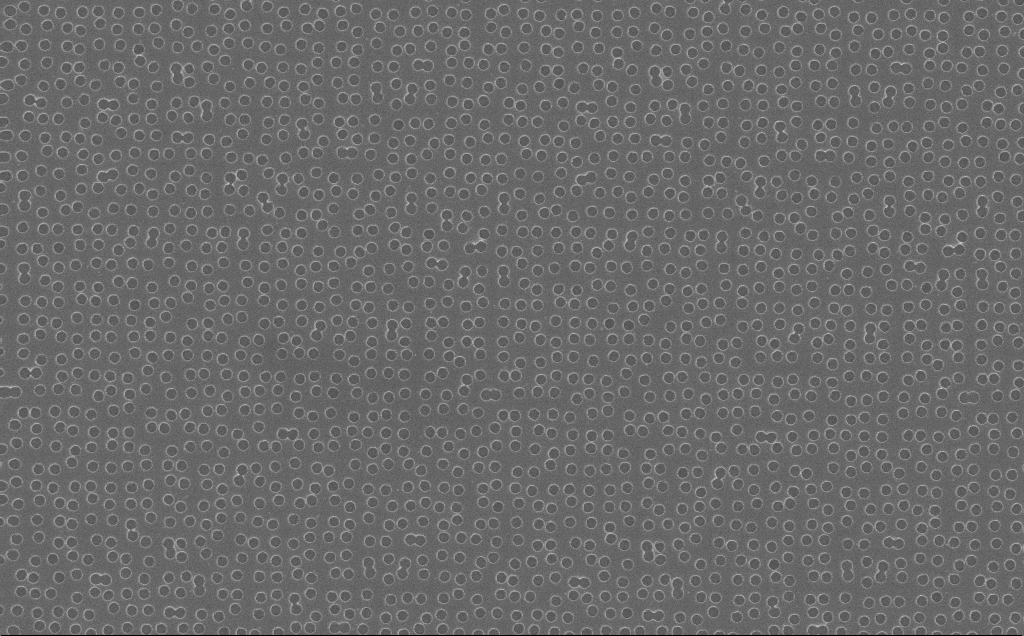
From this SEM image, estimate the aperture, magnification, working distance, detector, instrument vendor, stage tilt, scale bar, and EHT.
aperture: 30 µm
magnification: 16.92 K X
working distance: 7 mm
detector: InLens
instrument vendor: Zeiss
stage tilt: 0°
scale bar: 2000 nm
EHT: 10 kV